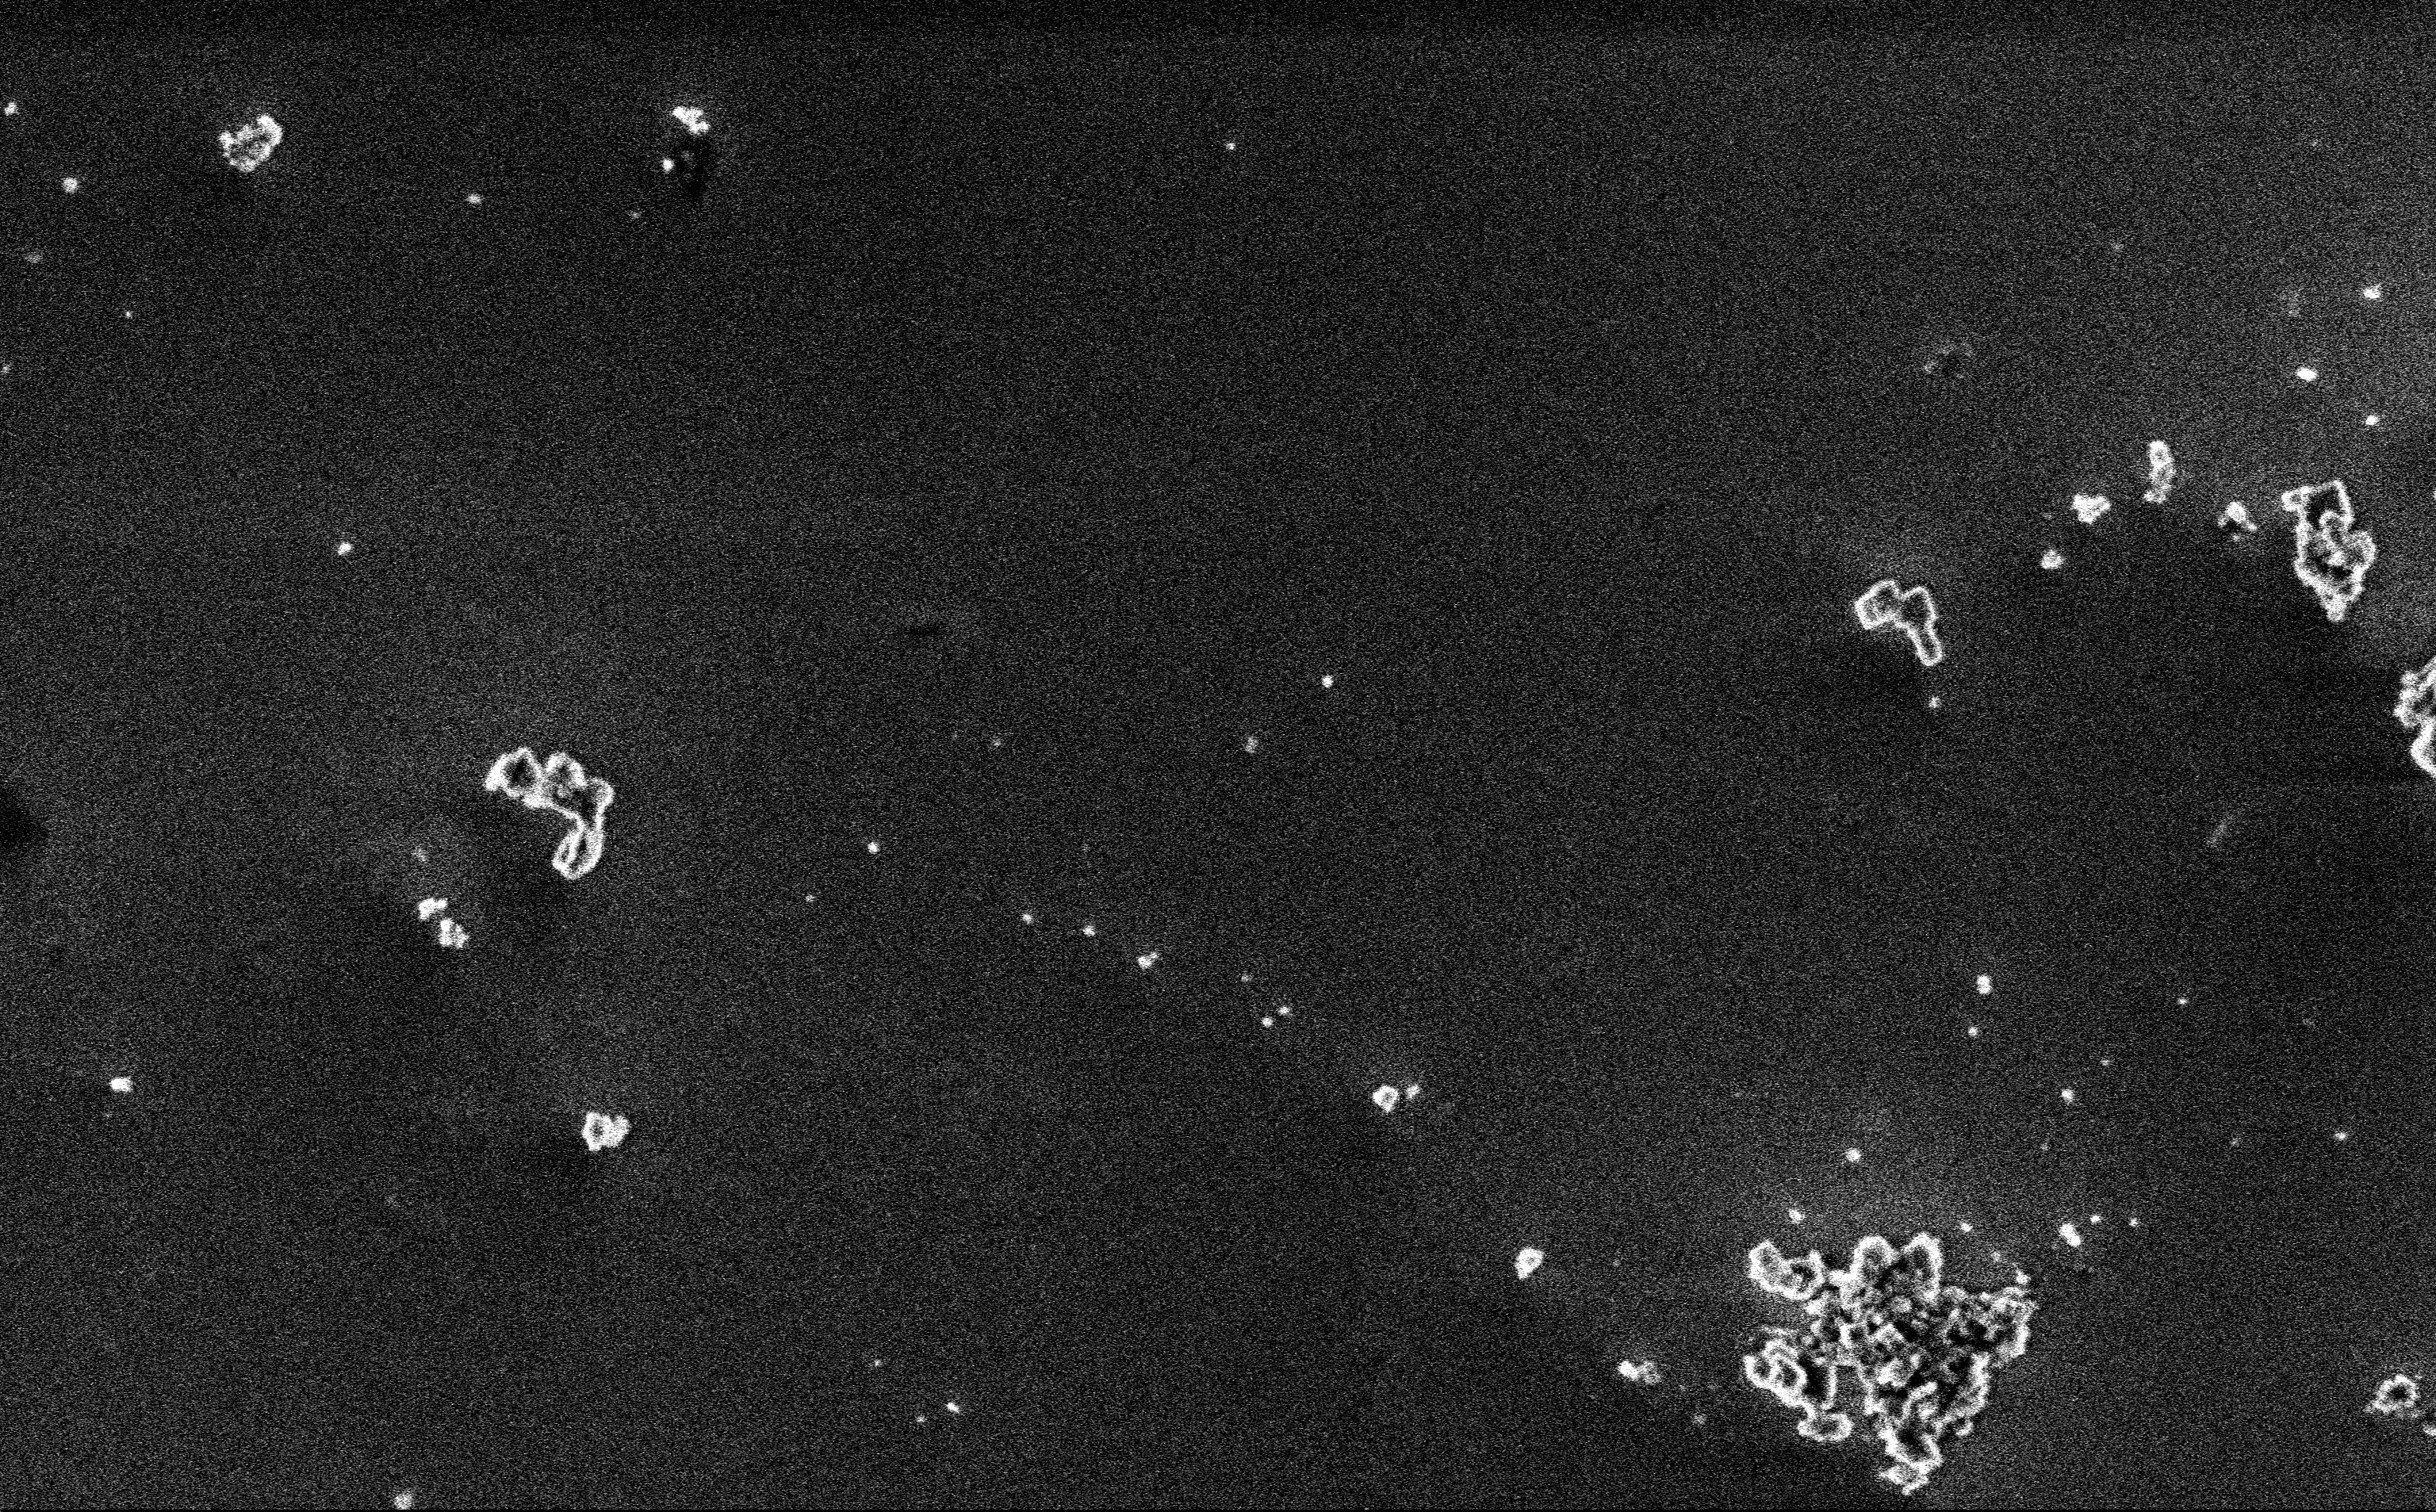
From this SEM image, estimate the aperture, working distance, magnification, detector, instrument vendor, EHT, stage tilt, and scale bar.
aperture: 30 µm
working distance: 3 mm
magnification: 12.85 K X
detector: InLens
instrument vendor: Zeiss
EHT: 3 kV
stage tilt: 0°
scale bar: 2000 nm